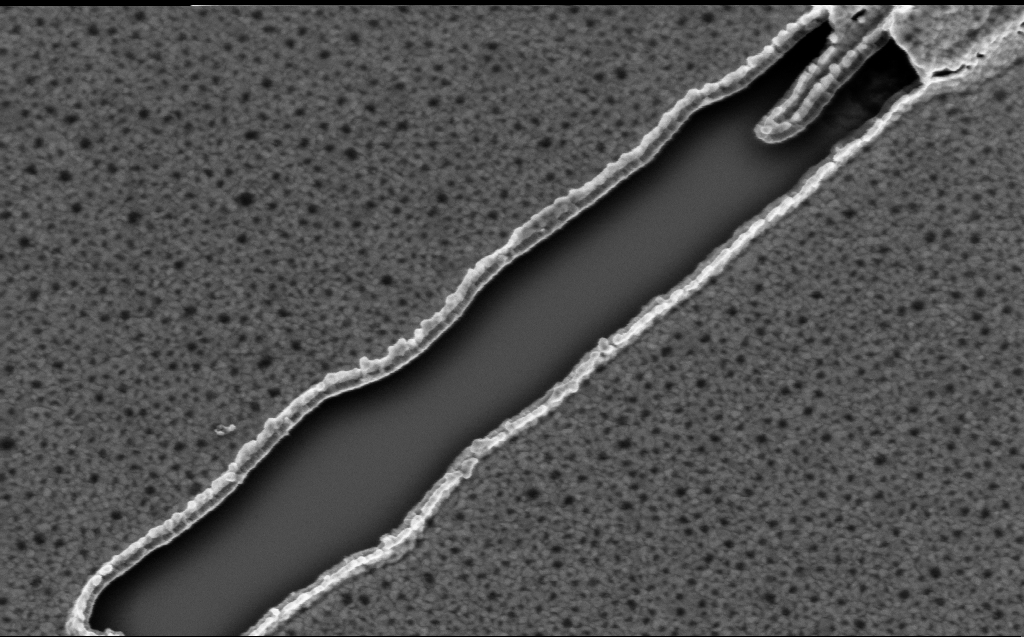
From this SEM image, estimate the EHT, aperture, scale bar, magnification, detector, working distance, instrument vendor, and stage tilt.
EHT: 3 kV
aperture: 30 µm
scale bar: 1000 nm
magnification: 71.31 K X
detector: InLens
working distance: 4 mm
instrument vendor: Zeiss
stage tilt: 20°